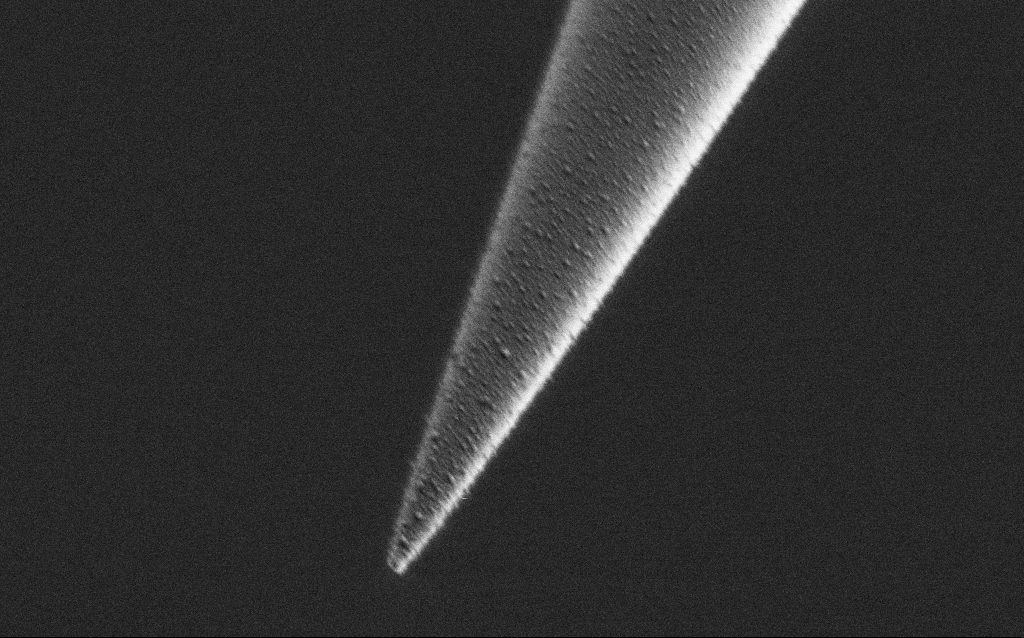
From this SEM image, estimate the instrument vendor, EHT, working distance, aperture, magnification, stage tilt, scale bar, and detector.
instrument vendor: Zeiss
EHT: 1 kV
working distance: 7.4 mm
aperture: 30 µm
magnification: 50 K X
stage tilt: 45°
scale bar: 1000 nm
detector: SE2